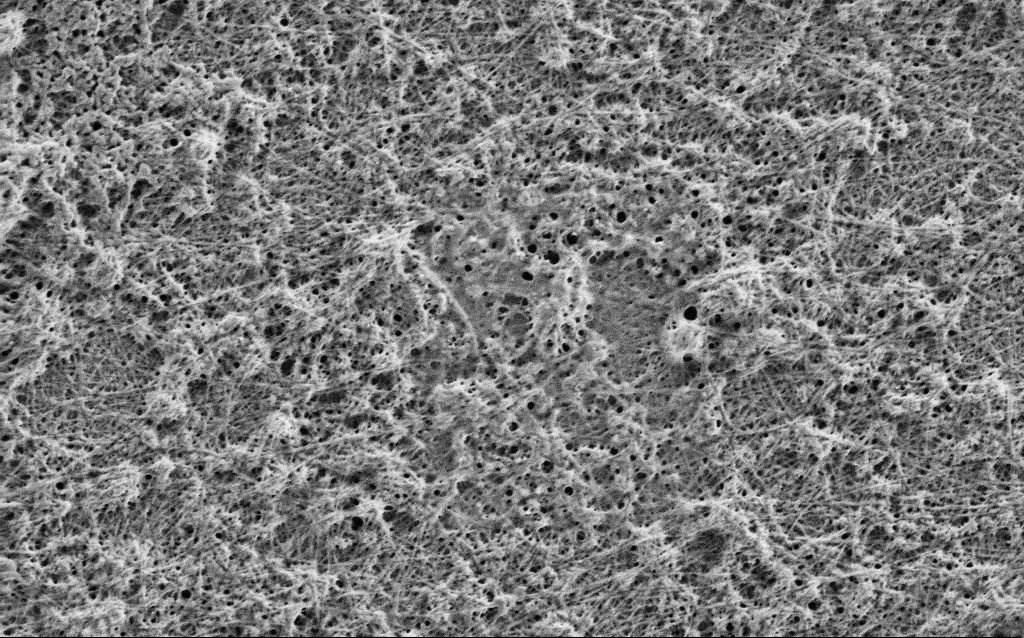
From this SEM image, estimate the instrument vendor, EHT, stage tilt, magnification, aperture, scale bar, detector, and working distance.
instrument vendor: Zeiss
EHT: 1 kV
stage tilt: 0°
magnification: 25 K X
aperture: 30 µm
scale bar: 2000 nm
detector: SE2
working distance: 4 mm